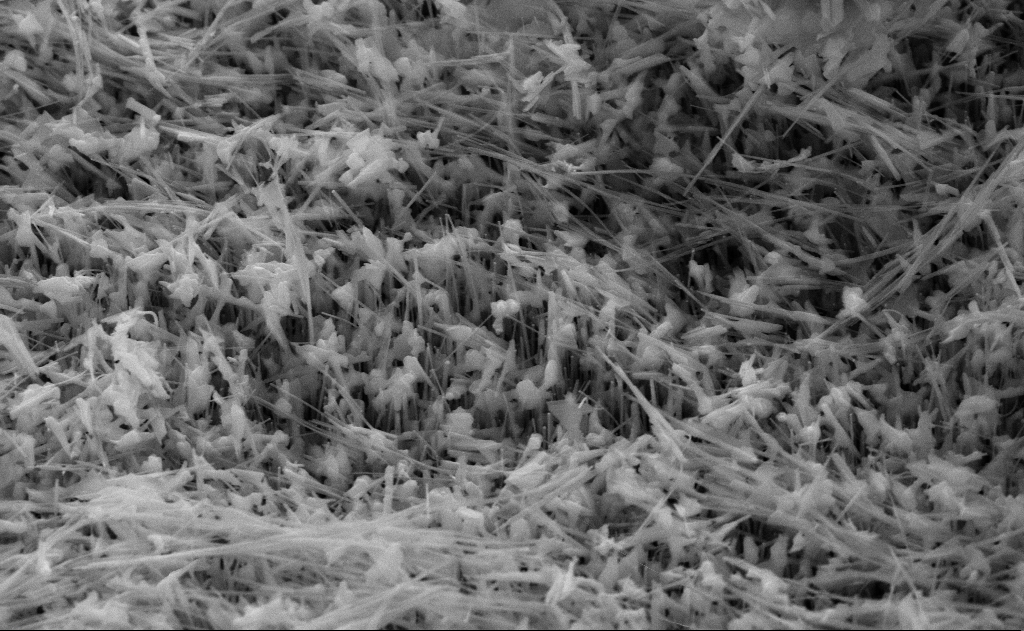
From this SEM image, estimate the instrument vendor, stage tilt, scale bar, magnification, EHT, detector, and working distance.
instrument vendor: Zeiss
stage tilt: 45°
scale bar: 1000 nm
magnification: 40 K X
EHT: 10 kV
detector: SE2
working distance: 11 mm